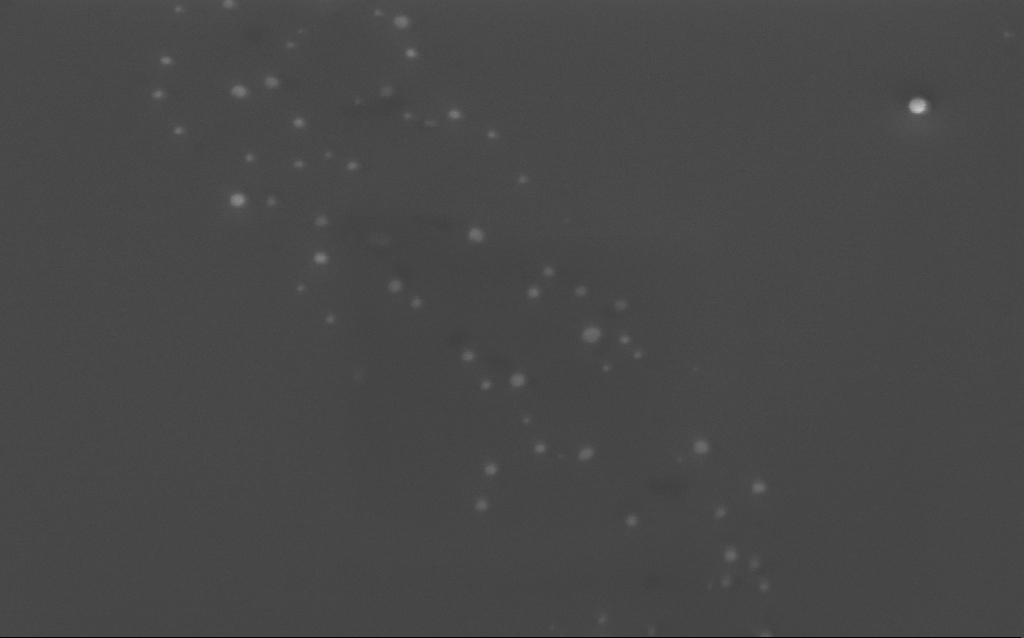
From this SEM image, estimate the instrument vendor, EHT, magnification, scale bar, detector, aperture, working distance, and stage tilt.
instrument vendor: Zeiss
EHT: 3 kV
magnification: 85.74 K X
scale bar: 200 nm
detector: InLens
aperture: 30 µm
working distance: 5 mm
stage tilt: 40°